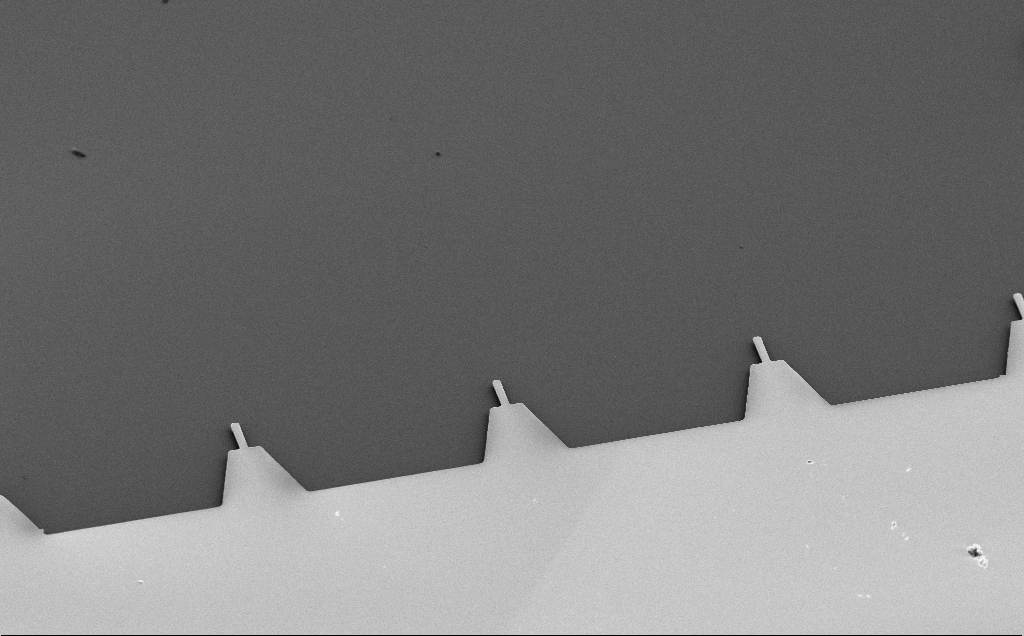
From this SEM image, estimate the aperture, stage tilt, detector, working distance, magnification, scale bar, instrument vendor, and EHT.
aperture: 30 µm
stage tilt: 50°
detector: SE2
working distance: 10 mm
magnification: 0.62 K X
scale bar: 100000 nm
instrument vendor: Zeiss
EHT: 5 kV